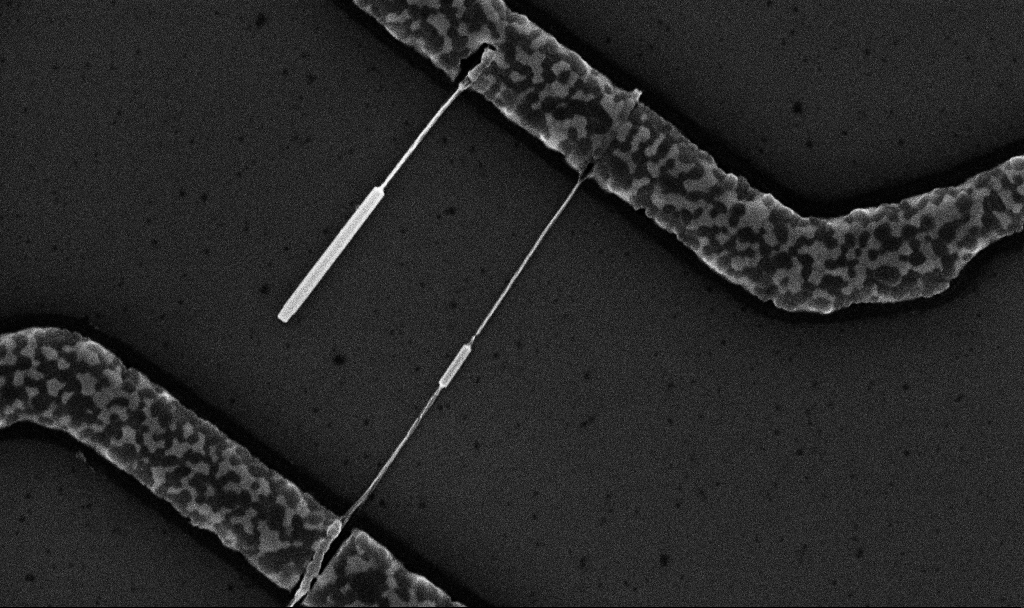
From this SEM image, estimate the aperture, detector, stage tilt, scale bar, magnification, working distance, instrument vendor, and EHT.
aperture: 30 µm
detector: InLens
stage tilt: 0°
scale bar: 1000 nm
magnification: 39.4 K X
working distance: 6.7 mm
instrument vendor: Zeiss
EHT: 10 kV